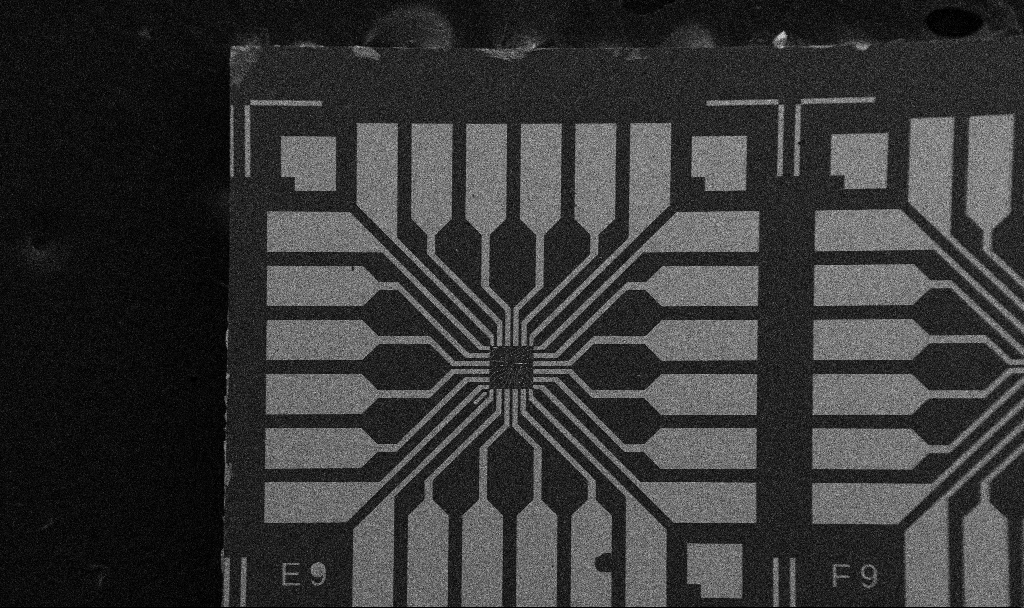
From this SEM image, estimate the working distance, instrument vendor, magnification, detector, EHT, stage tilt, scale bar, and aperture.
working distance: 10.7 mm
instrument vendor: Zeiss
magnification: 0.1 K X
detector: SE2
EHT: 5 kV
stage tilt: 0°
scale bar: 200000 nm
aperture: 30 µm